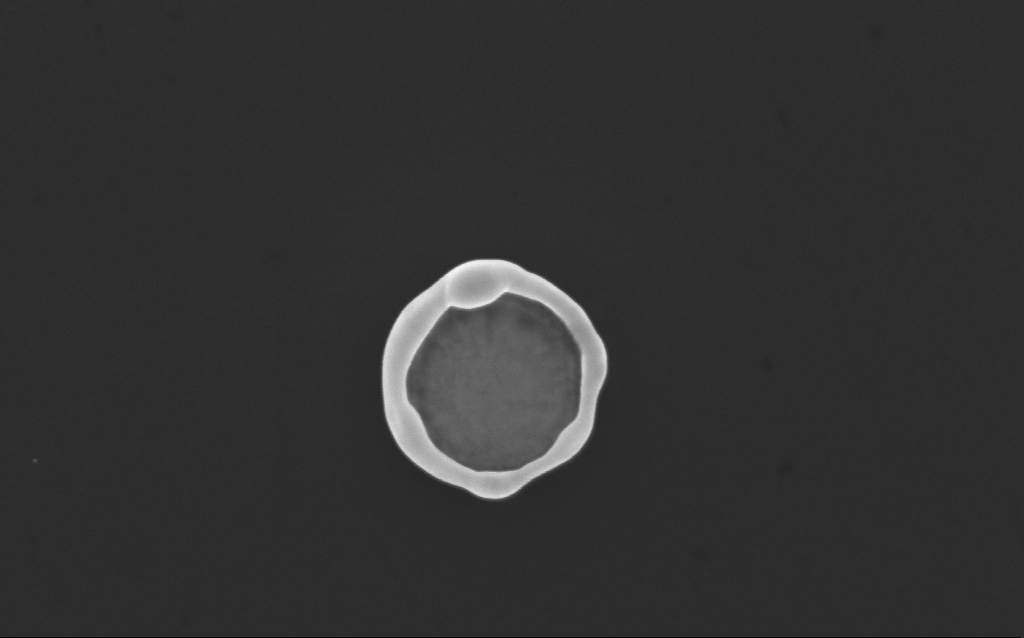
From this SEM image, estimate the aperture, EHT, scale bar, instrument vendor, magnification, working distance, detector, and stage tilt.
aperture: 30 µm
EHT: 10 kV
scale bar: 1000 nm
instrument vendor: Zeiss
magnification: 67.26 K X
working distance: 4 mm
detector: InLens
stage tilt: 0°